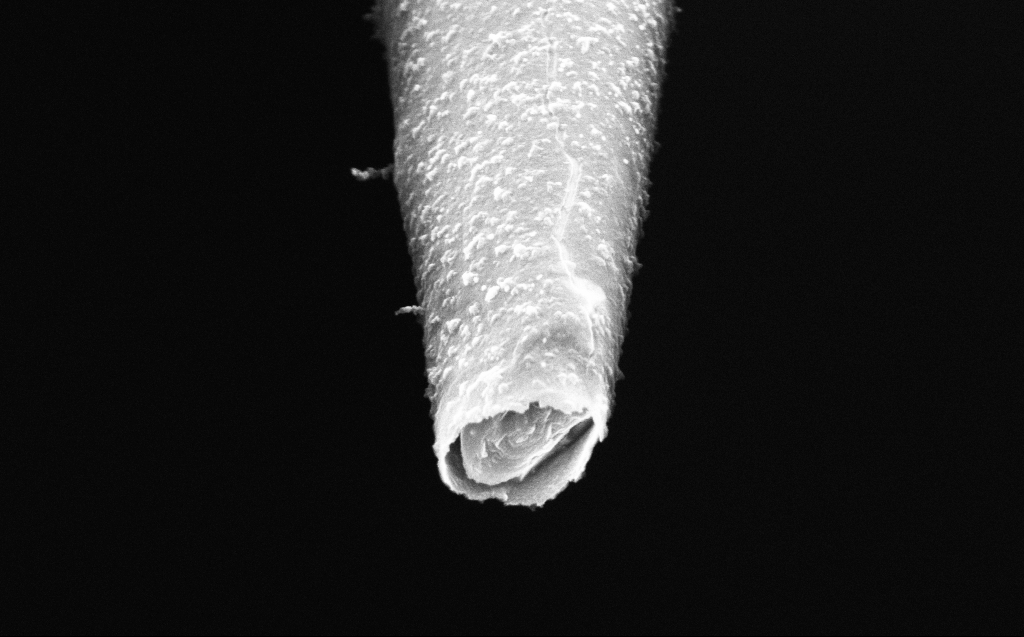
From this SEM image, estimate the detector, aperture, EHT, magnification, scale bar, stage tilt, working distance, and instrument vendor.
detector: InLens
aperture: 30 µm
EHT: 4 kV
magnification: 100 K X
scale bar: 200 nm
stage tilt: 45°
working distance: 4 mm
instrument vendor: Zeiss